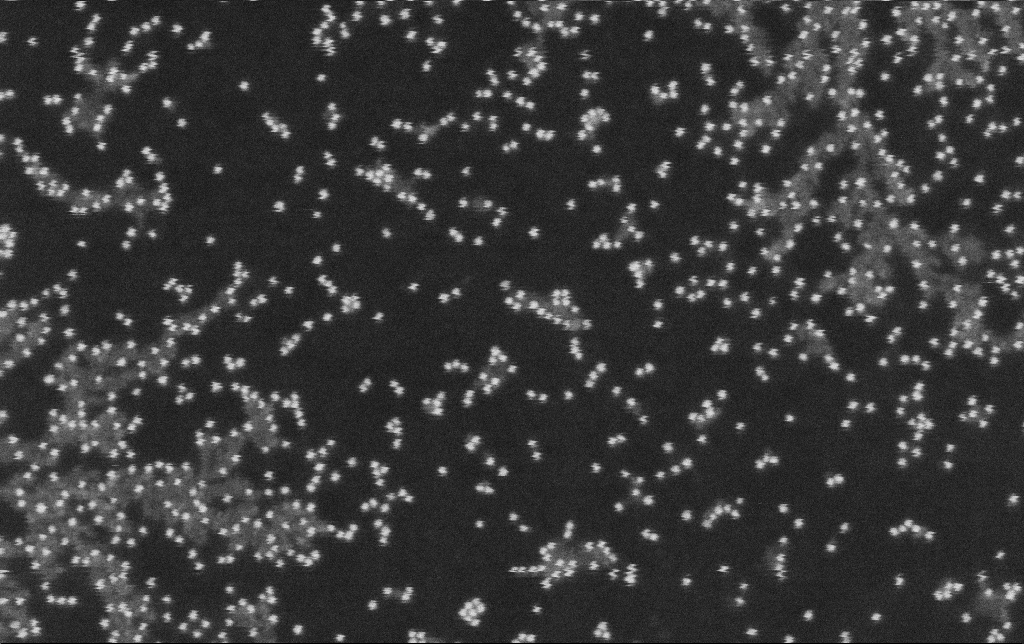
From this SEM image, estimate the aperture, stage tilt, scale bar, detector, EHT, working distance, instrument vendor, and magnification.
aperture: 30 µm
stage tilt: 0°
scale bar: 200 nm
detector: SE2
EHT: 10 kV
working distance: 11.3 mm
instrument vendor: Zeiss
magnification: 216.33 K X